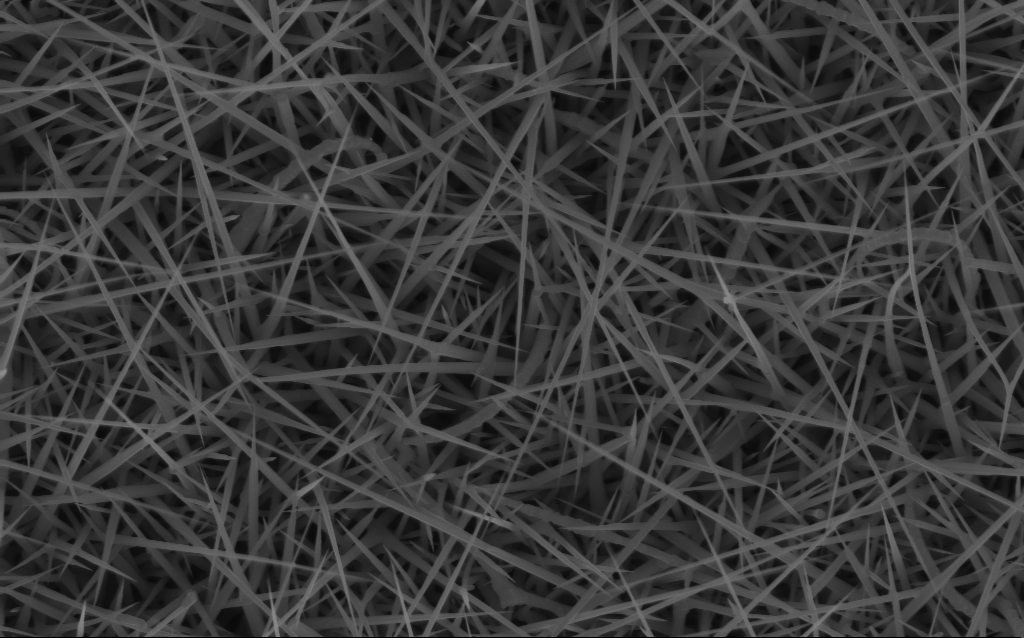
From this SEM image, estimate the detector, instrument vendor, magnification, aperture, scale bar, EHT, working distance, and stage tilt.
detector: InLens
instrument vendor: Zeiss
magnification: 20 K X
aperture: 30 µm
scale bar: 2000 nm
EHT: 10 kV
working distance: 7 mm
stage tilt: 0°